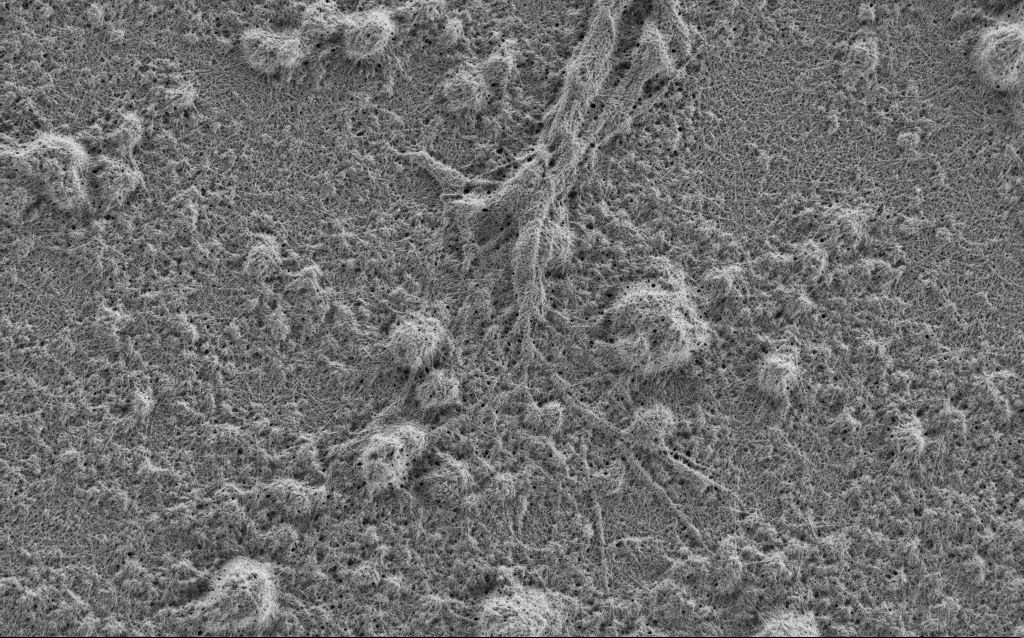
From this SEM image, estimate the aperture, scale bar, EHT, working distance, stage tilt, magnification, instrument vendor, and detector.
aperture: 30 µm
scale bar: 2000 nm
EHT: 0.9 kV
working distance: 4 mm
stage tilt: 0°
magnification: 10 K X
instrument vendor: Zeiss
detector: SE2